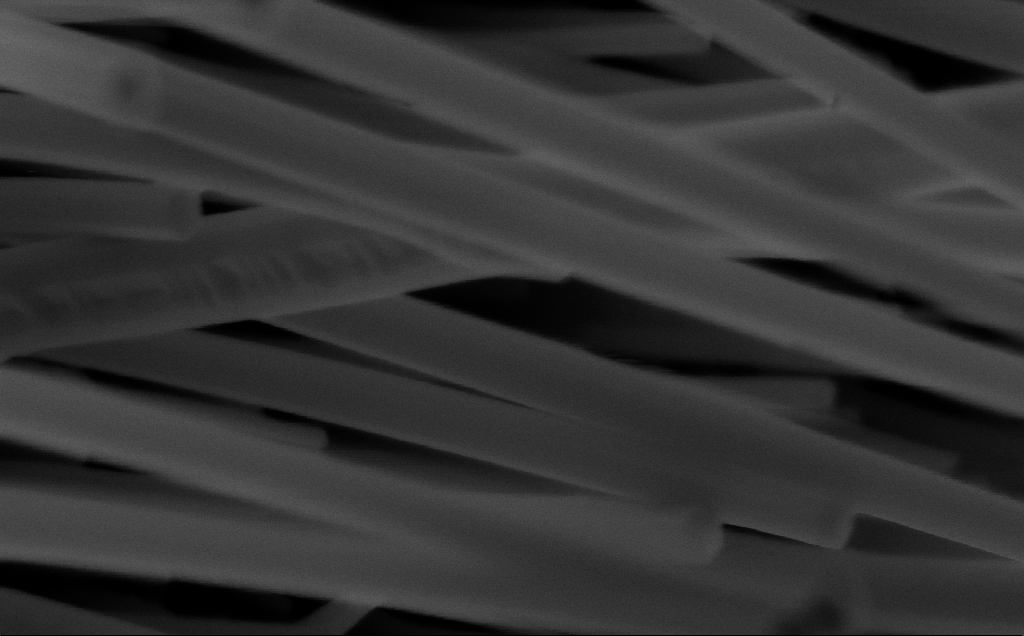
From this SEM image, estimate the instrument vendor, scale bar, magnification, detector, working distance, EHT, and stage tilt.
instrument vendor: Zeiss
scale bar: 200 nm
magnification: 182.09 K X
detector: InLens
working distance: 6 mm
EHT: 5 kV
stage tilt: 0°